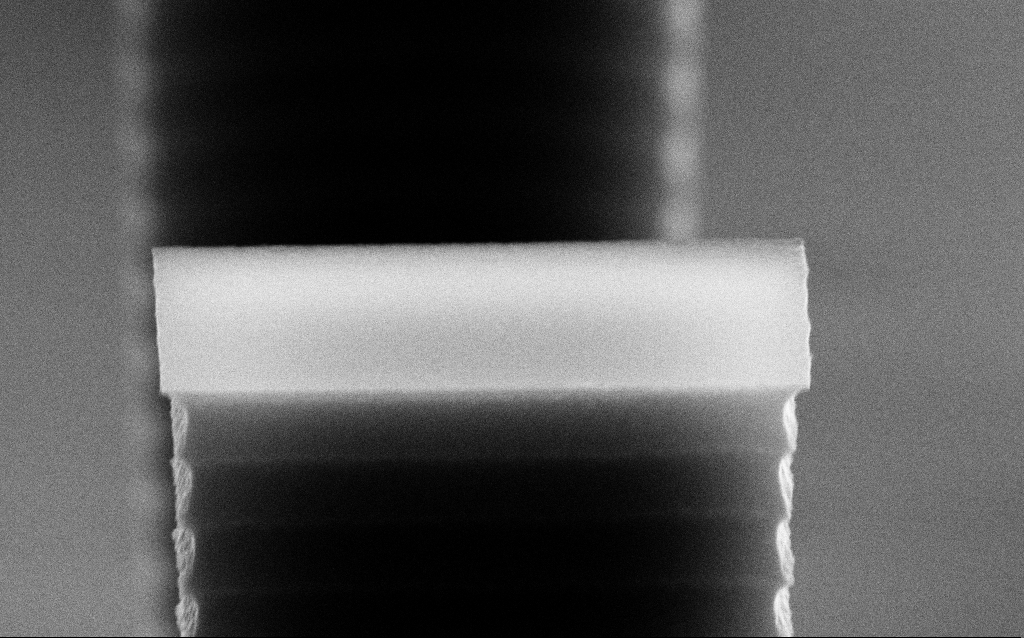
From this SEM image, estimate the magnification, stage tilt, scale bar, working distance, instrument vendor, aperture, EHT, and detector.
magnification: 81.13 K X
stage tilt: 70°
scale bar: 200 nm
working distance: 7.5 mm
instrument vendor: Zeiss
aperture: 30 µm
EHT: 10 kV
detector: SE2